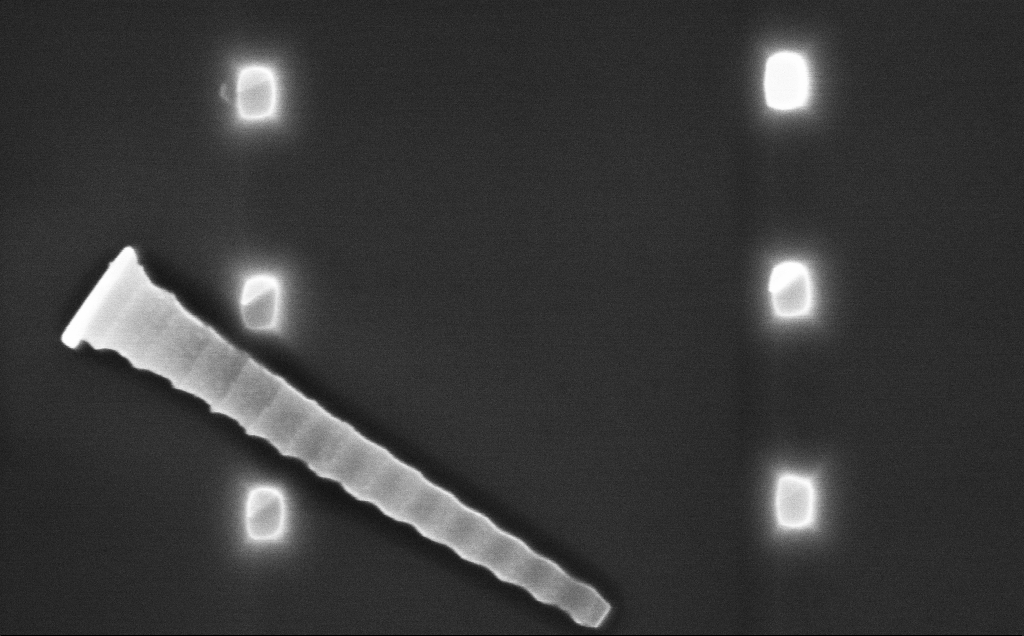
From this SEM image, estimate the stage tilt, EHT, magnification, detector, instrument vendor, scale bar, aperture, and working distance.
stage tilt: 0°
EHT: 10 kV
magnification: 39.16 K X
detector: InLens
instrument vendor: Zeiss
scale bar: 1000 nm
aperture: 30 µm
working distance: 9 mm